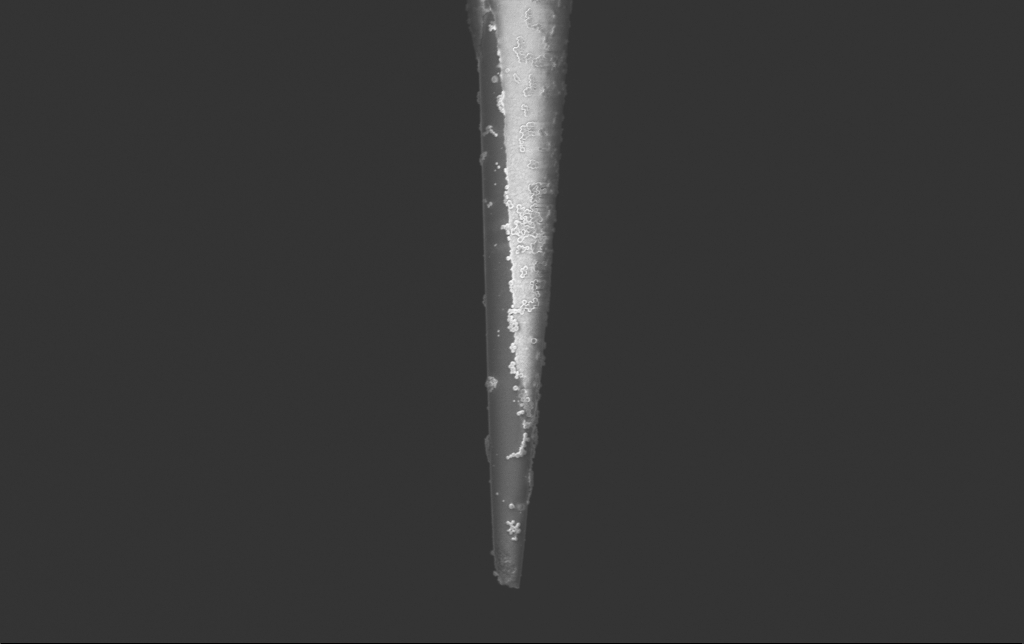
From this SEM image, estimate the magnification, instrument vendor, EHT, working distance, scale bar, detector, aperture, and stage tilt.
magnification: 15 K X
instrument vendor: Zeiss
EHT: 2 kV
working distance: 5.9 mm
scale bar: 1000 nm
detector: InLens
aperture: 30 µm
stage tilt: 0°